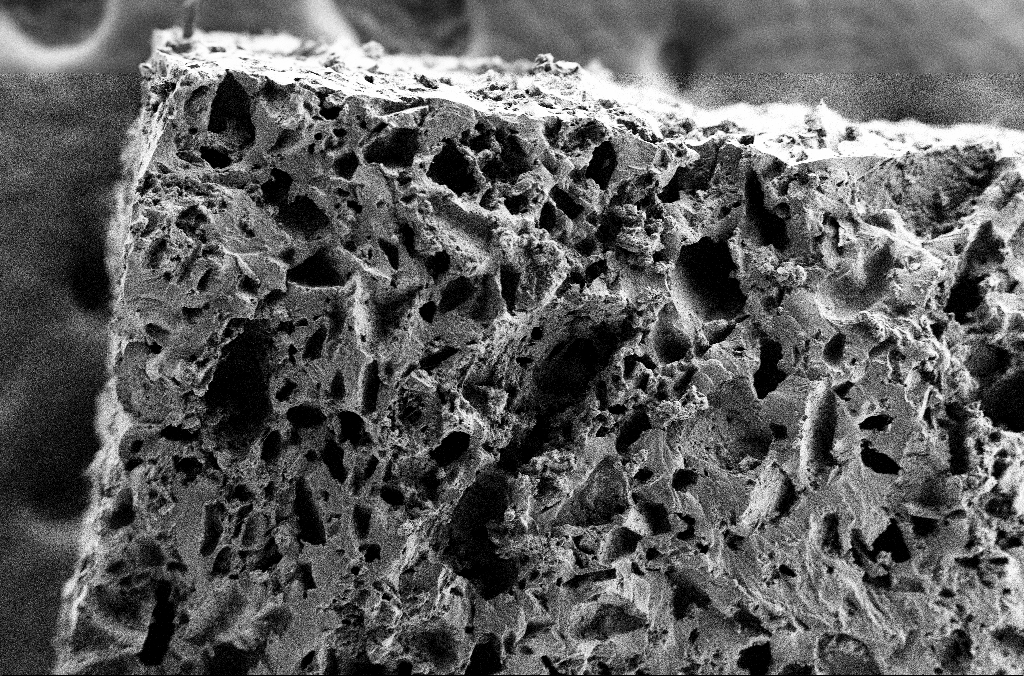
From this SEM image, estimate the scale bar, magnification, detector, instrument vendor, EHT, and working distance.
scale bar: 100000 nm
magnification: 0.243 K X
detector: SE2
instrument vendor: Zeiss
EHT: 2 kV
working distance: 3 mm